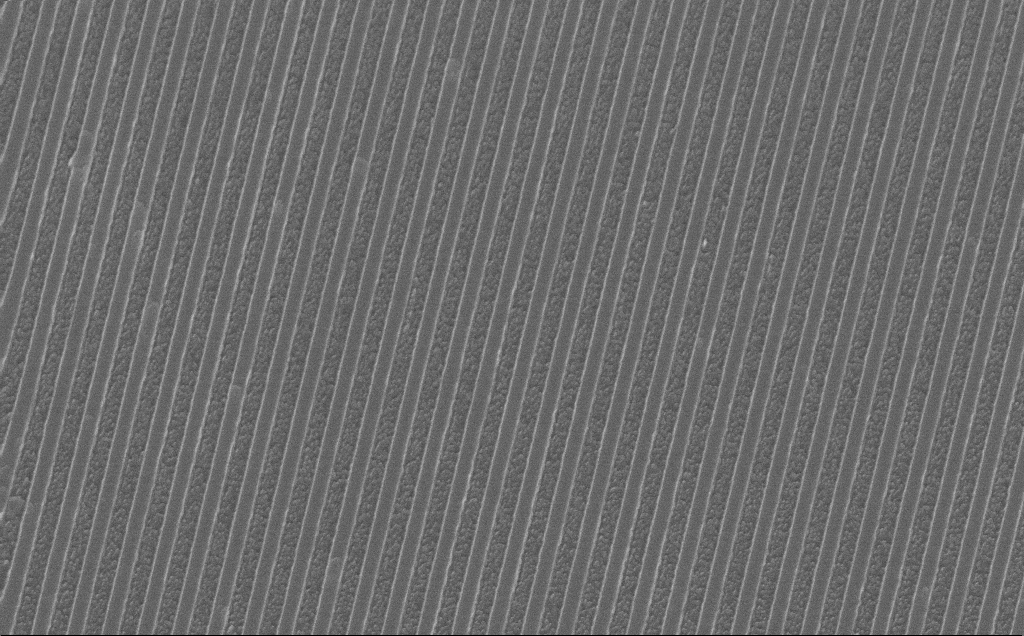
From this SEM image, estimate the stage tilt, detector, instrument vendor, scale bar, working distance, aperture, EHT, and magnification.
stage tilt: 38°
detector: InLens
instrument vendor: Zeiss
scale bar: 2000 nm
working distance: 6 mm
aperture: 30 µm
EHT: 10 kV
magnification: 24.95 K X